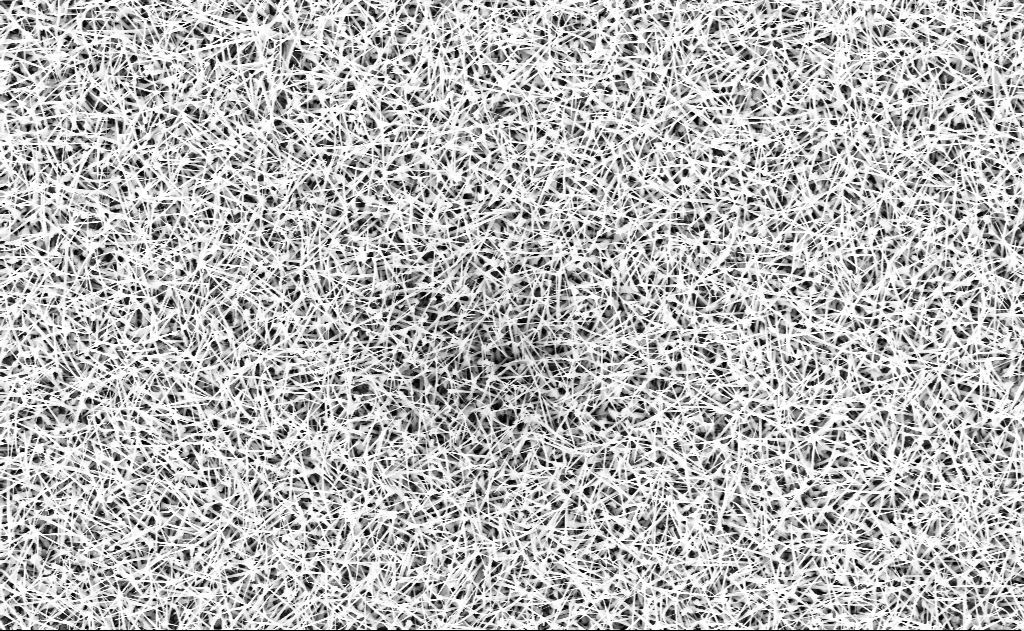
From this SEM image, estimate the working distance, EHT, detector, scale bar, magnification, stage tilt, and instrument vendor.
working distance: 14 mm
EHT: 10 kV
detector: InLens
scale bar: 2000 nm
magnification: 10 K X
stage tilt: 0°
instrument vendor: Zeiss